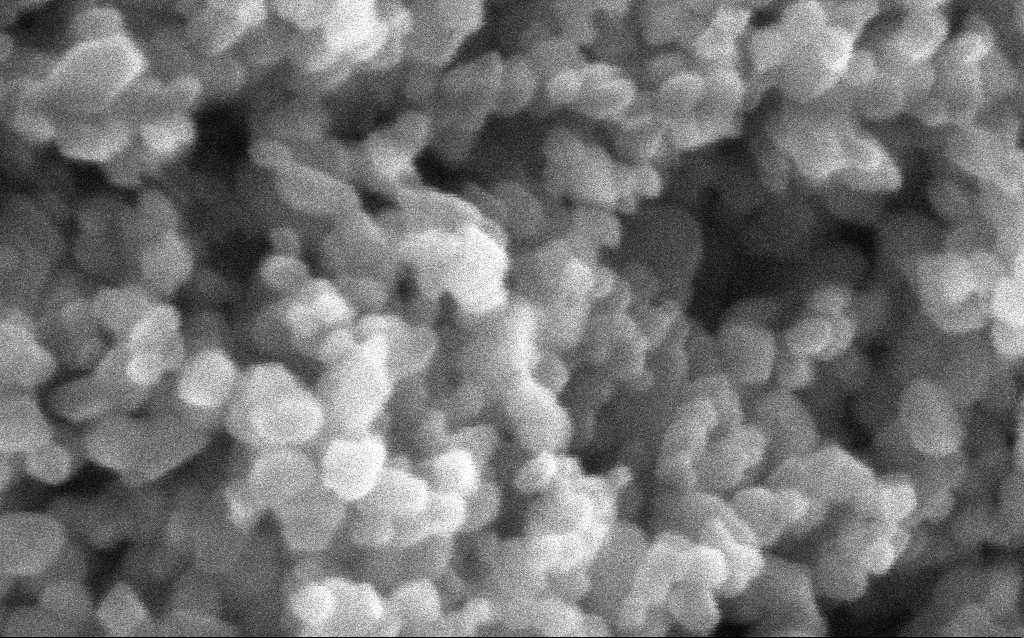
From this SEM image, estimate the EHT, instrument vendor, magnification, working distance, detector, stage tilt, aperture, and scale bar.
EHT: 5 kV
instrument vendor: Zeiss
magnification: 716 K X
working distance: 2.9 mm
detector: InLens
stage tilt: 0°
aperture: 30 µm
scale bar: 100 nm